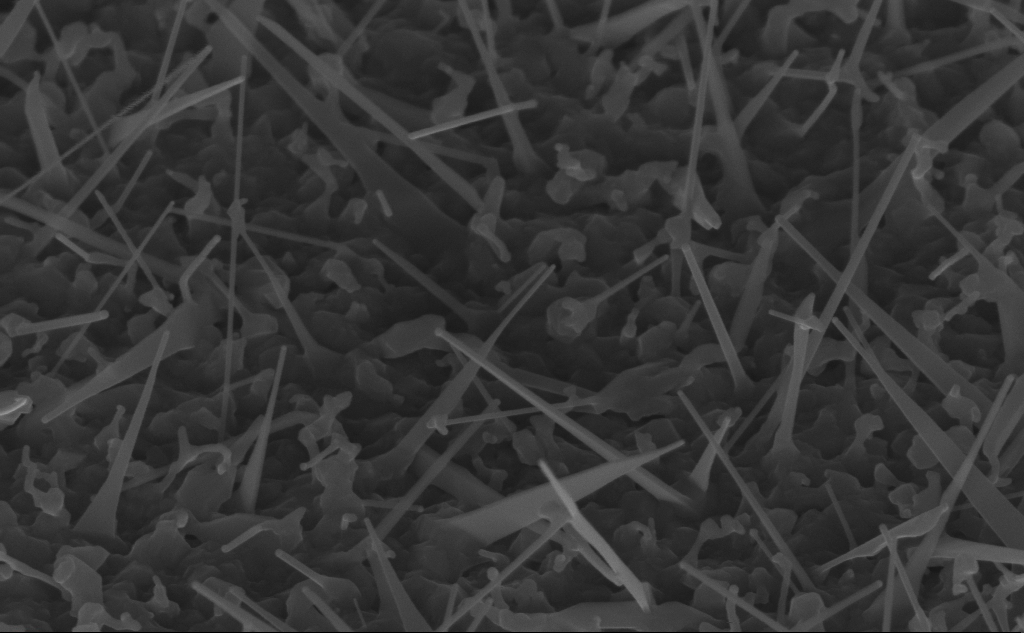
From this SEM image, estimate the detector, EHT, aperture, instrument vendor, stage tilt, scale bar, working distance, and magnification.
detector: InLens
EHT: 10 kV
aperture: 30 µm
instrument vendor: Zeiss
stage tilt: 45°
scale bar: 200 nm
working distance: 8 mm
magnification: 80 K X